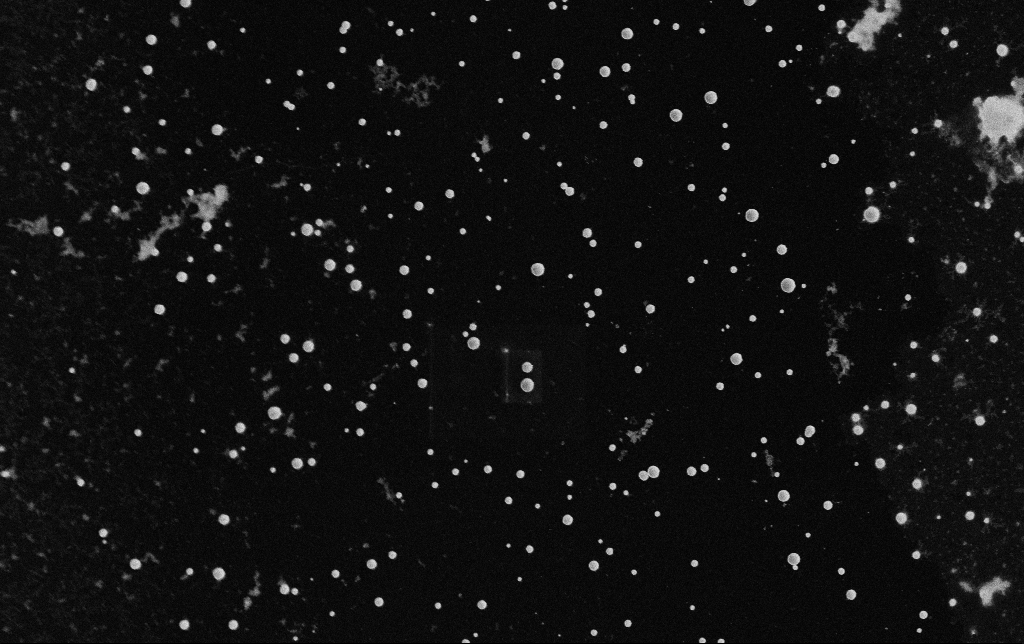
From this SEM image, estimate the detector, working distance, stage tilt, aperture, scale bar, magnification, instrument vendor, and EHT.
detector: SE2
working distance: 11.3 mm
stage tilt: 0°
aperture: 30 µm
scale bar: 1000 nm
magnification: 19.08 K X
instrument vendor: Zeiss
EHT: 8 kV